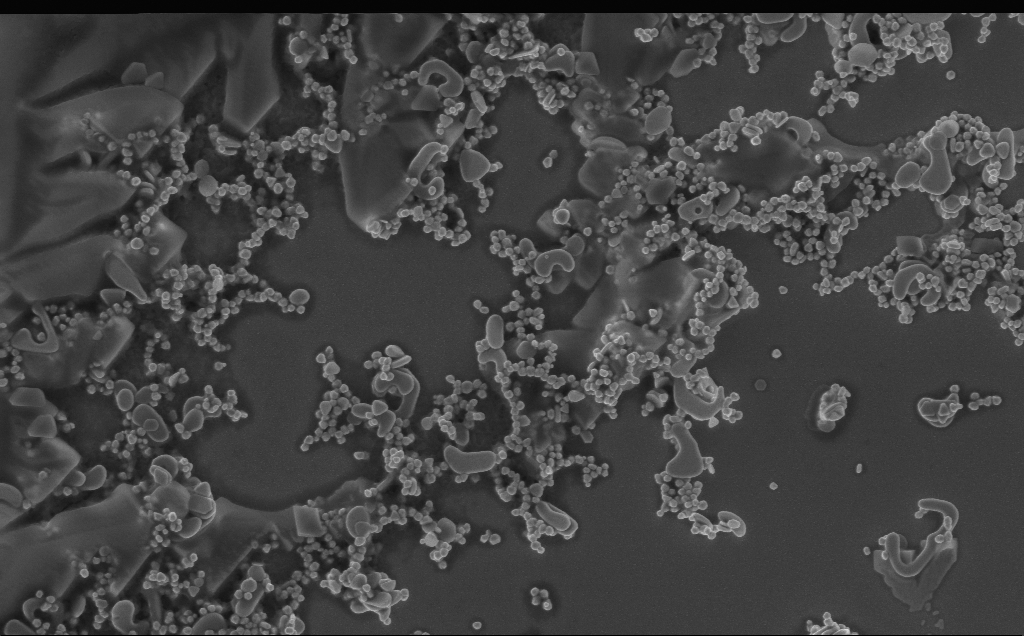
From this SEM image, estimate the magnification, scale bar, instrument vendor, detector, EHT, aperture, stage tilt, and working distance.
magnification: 22.24 K X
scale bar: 1000 nm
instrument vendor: Zeiss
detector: InLens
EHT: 5 kV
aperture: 30 µm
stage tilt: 0°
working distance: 3 mm